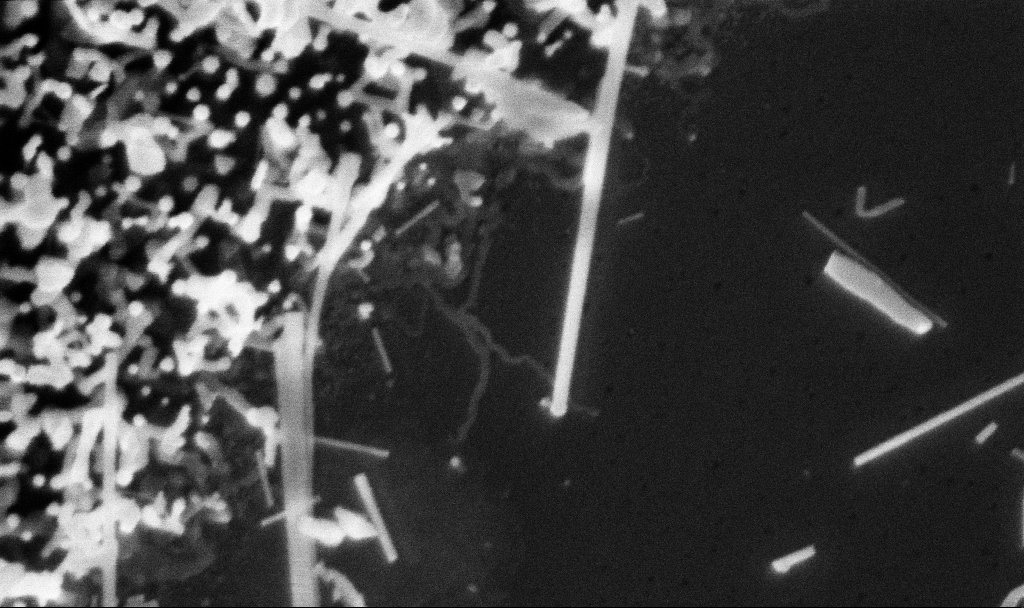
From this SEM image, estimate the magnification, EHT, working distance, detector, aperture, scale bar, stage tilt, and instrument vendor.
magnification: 168.01 K X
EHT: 3 kV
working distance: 3.1 mm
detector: InLens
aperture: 30 µm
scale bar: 200 nm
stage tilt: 0°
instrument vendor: Zeiss